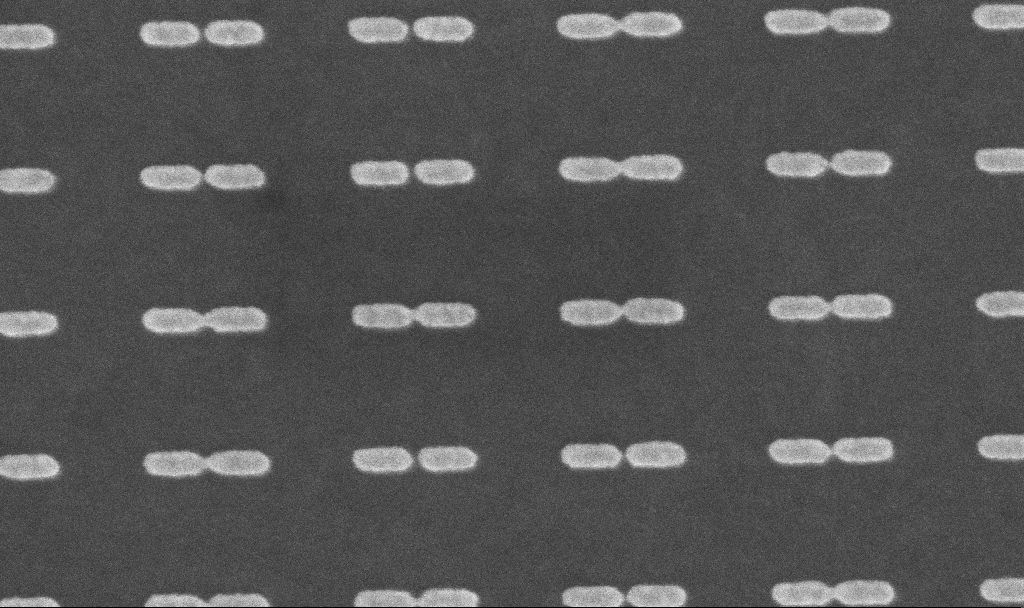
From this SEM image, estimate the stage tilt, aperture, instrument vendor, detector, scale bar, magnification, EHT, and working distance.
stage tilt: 0°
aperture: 30 µm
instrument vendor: Zeiss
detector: InLens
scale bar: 200 nm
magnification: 175.6 K X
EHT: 5 kV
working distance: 6 mm